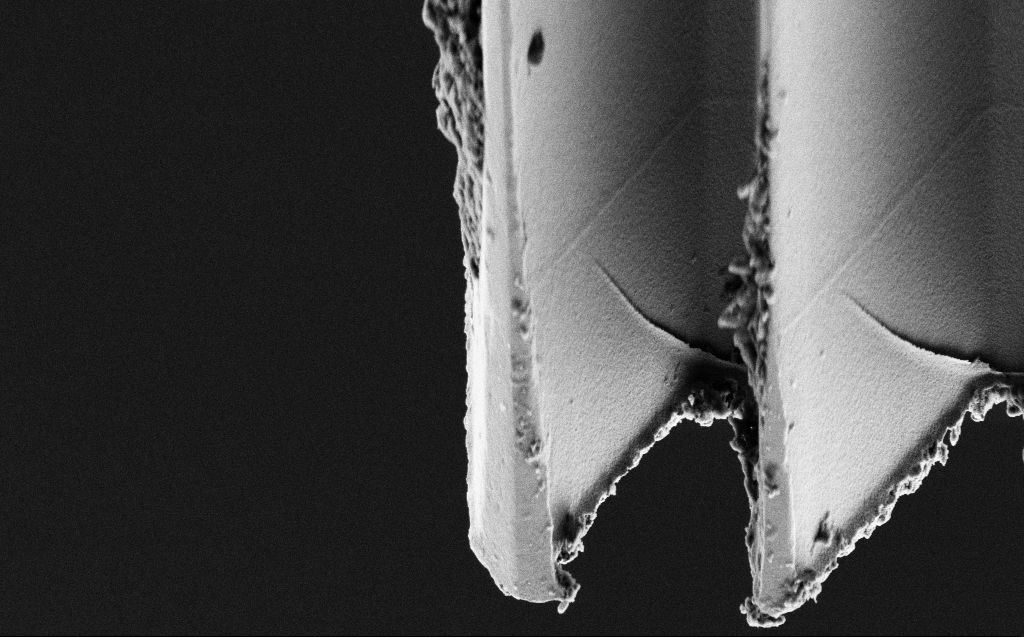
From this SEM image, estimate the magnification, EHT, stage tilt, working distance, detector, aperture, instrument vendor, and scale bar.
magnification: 9.15 K X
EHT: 3 kV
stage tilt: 45°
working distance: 8 mm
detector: SE2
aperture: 30 µm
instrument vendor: Zeiss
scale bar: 2000 nm